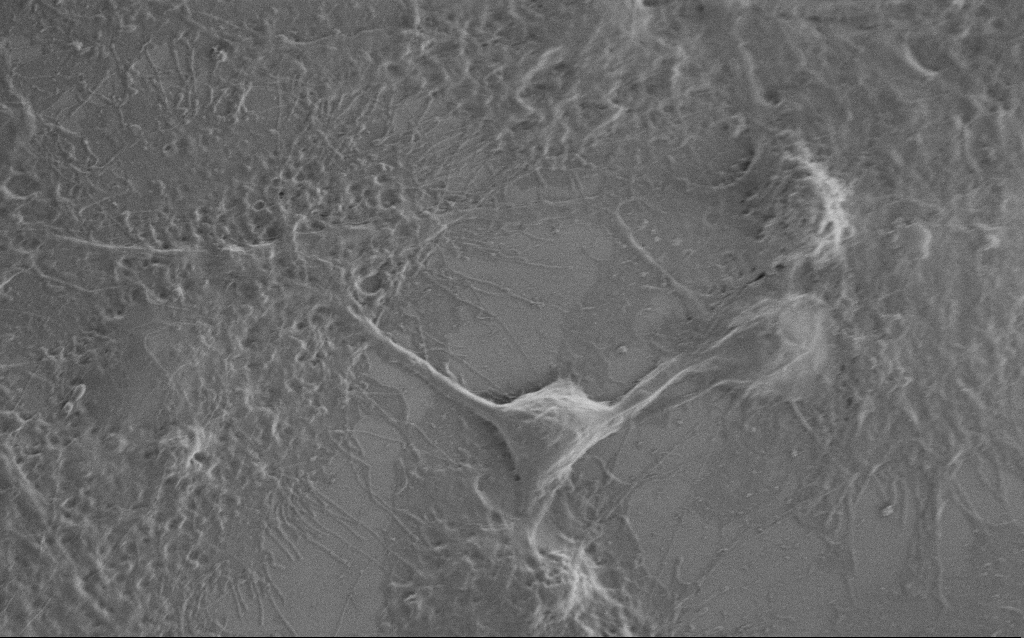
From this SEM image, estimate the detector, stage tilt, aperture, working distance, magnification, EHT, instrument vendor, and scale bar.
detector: SE2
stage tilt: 0°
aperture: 30 µm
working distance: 6.1 mm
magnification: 5 K X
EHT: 0.8 kV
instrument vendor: Zeiss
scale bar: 10000 nm